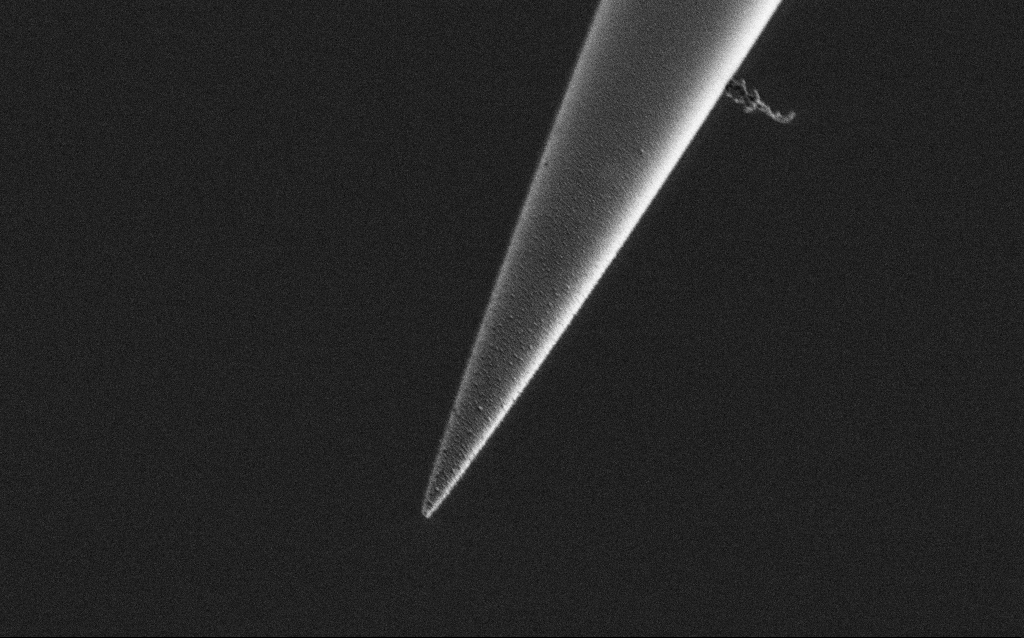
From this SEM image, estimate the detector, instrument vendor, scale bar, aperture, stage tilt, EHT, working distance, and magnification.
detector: SE2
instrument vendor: Zeiss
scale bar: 2000 nm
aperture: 30 µm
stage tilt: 45°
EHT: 1 kV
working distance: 7.4 mm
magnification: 25 K X